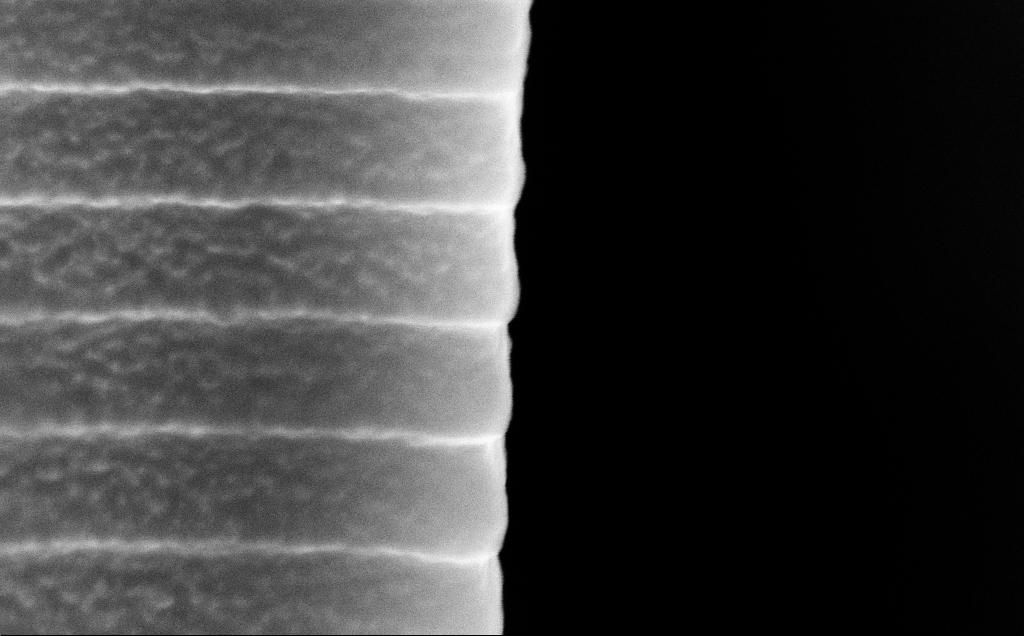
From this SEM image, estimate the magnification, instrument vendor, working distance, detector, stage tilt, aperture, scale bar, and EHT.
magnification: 117.84 K X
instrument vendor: Zeiss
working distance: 5 mm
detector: InLens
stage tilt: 45°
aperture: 30 µm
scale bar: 200 nm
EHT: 10 kV